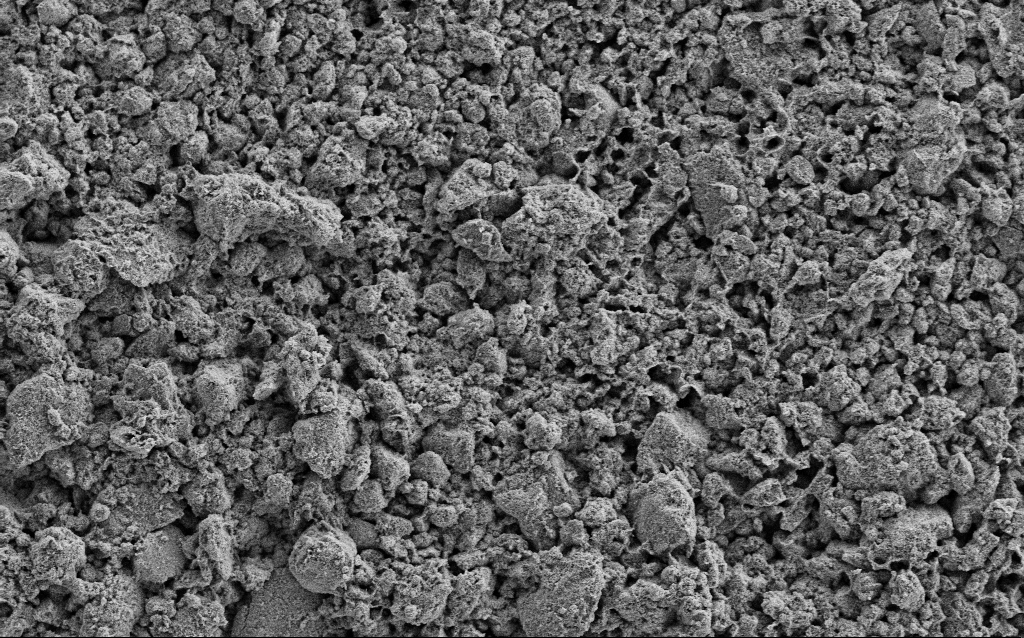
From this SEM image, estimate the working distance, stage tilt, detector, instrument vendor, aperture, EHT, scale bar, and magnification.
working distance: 4.4 mm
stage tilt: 0°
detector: SE2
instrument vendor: Zeiss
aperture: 30 µm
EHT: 5 kV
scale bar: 10000 nm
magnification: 1.23 K X